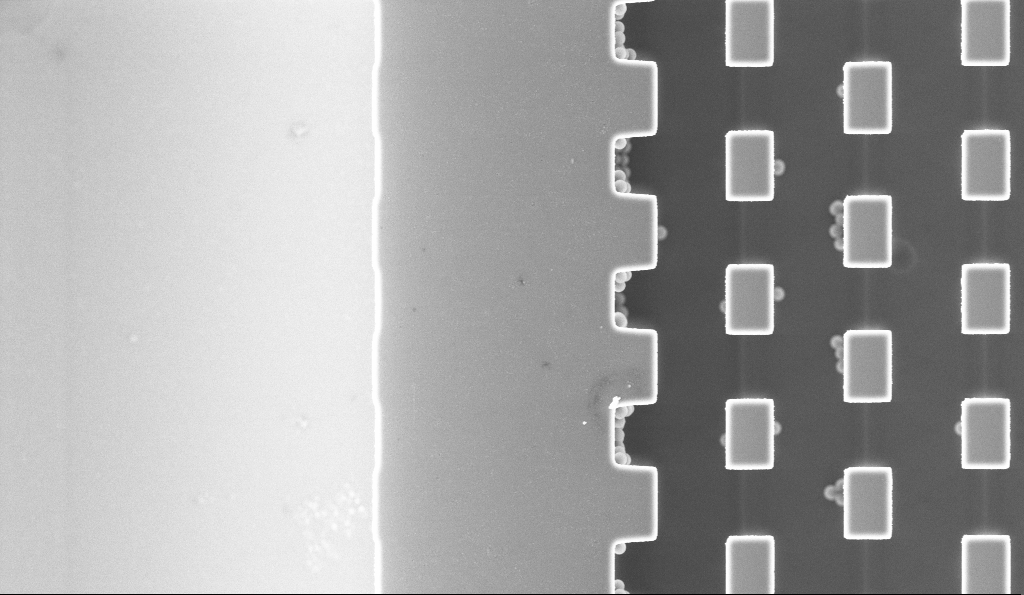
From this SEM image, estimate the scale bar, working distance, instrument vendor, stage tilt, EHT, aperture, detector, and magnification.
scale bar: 2000 nm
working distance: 3.1 mm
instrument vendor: Zeiss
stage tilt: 0°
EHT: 5 kV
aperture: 30 µm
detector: InLens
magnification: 8.34 K X